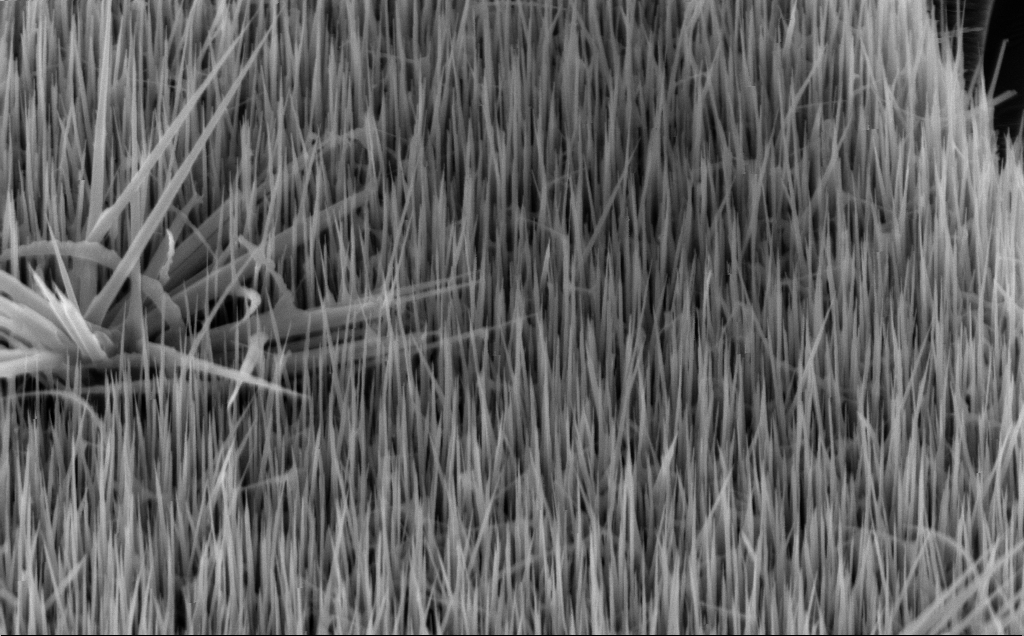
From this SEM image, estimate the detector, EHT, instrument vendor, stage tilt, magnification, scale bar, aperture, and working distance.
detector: InLens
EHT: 10 kV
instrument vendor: Zeiss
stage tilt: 45°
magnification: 40 K X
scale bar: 1000 nm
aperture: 30 µm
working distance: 8 mm